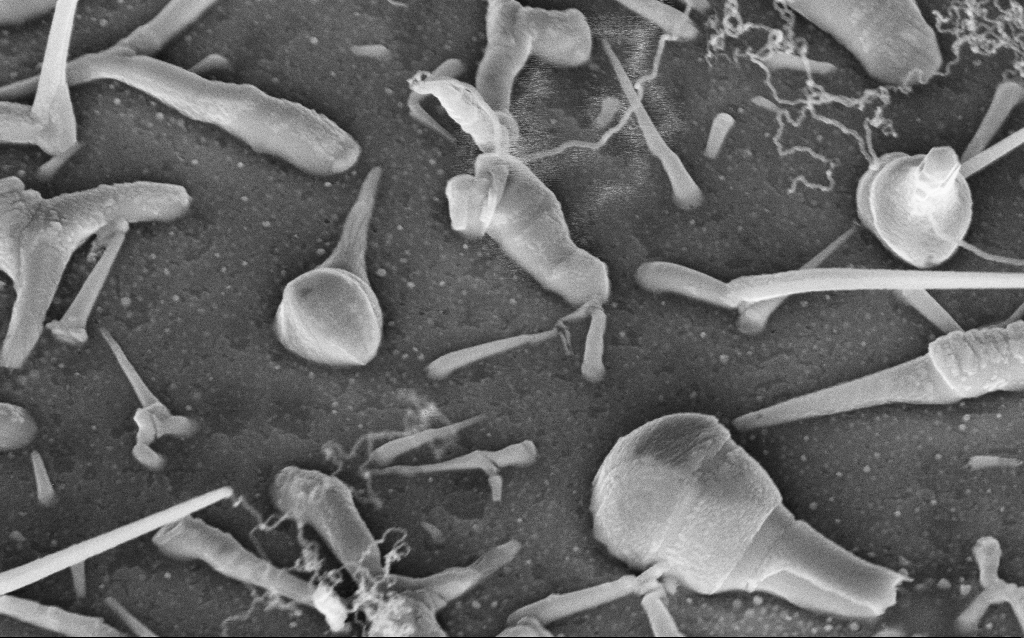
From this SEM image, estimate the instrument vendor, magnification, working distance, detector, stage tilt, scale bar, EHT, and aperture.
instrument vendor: Zeiss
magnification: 71.85 K X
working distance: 8 mm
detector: InLens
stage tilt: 42°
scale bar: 1000 nm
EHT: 5 kV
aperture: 30 µm